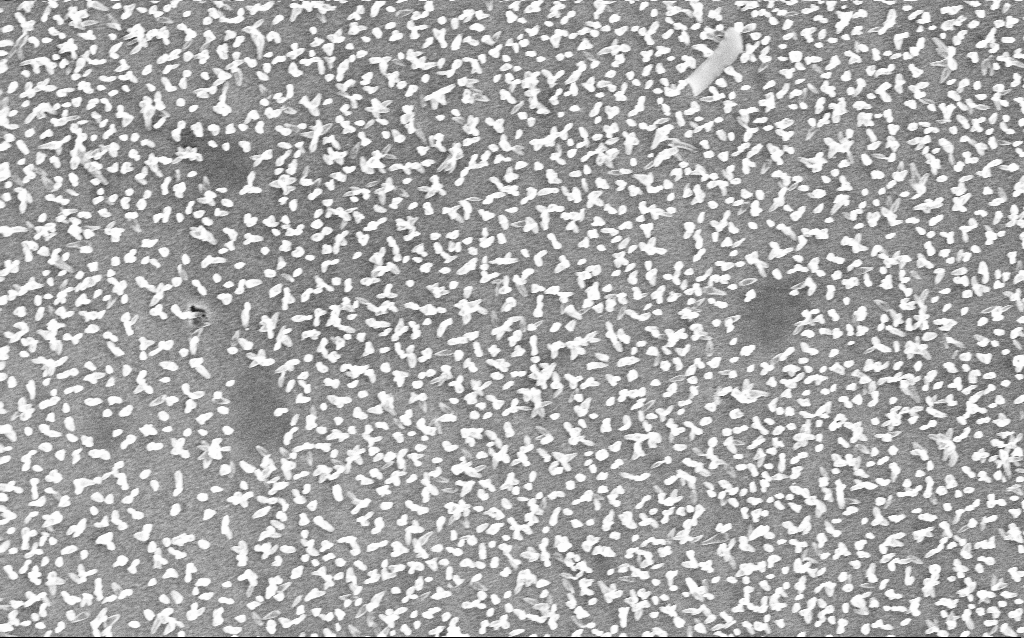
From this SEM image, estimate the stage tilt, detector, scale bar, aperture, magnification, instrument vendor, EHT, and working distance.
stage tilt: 0°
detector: InLens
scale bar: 2000 nm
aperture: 30 µm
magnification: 17.87 K X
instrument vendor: Zeiss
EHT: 6 kV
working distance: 6 mm